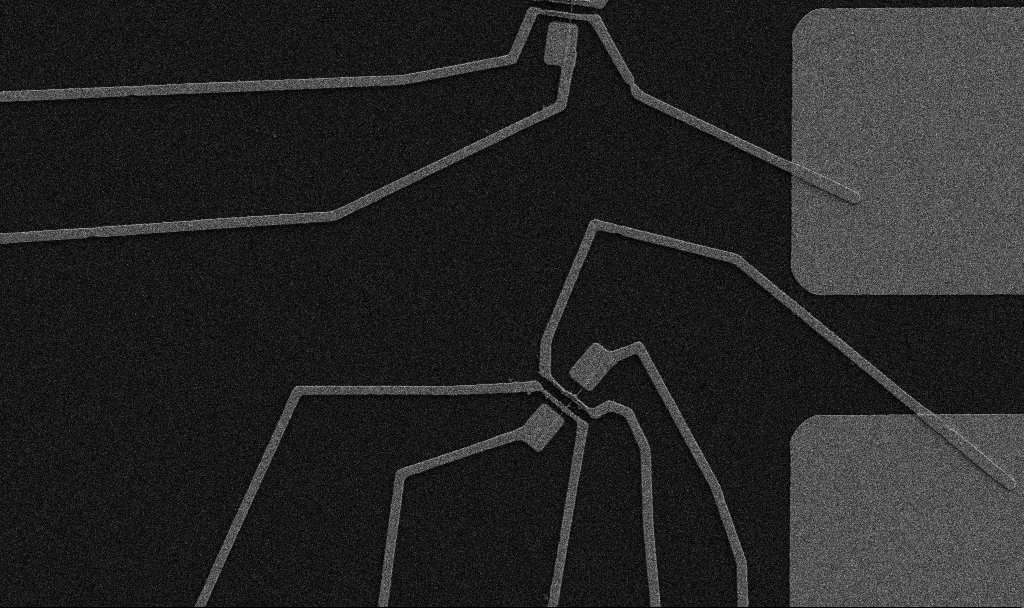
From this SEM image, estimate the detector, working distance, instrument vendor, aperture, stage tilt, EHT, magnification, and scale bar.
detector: SE2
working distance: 10.7 mm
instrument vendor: Zeiss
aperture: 30 µm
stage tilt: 0°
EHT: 5 kV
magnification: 5 K X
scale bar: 10000 nm